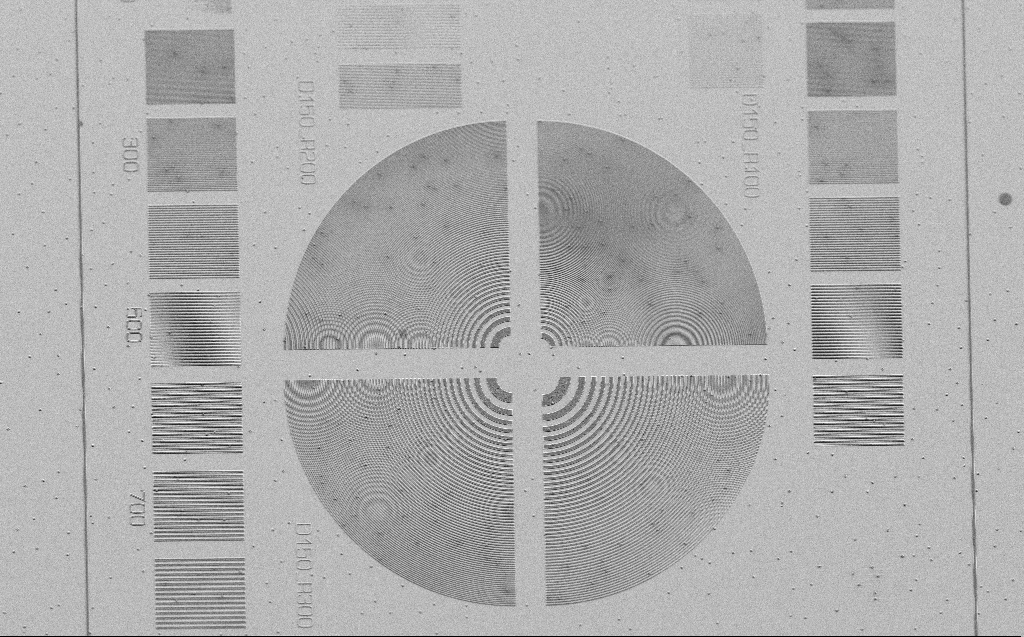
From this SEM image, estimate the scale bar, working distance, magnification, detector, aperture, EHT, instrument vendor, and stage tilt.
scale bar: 20000 nm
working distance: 6 mm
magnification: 1.12 K X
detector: SE2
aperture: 30 µm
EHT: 3 kV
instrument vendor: Zeiss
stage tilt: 30°